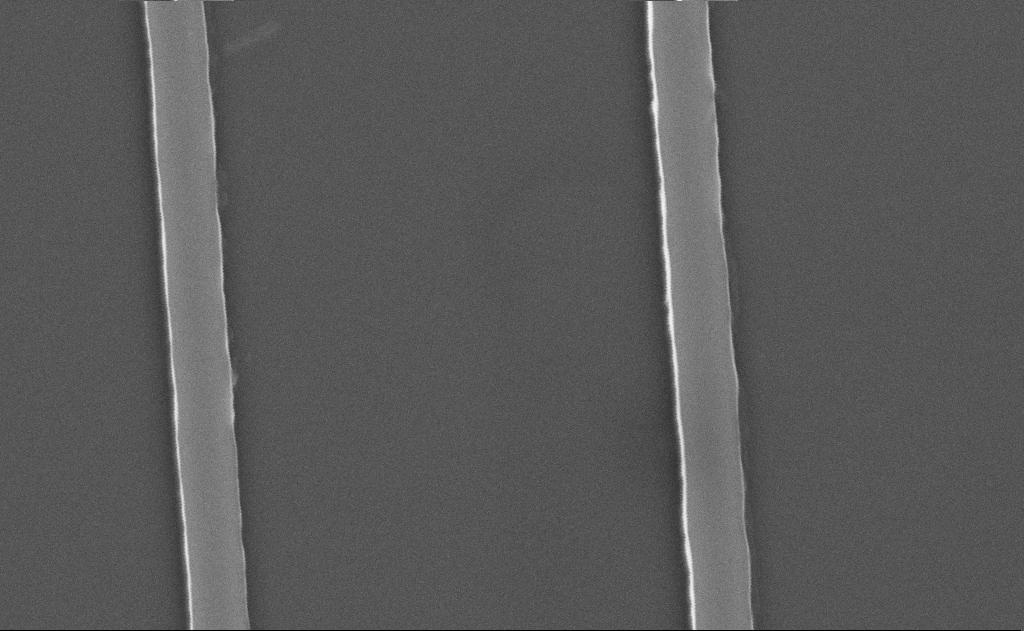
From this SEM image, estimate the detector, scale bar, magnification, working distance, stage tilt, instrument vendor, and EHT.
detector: SE2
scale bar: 1000 nm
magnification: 45.59 K X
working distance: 15 mm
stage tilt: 0°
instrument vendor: Zeiss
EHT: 5 kV